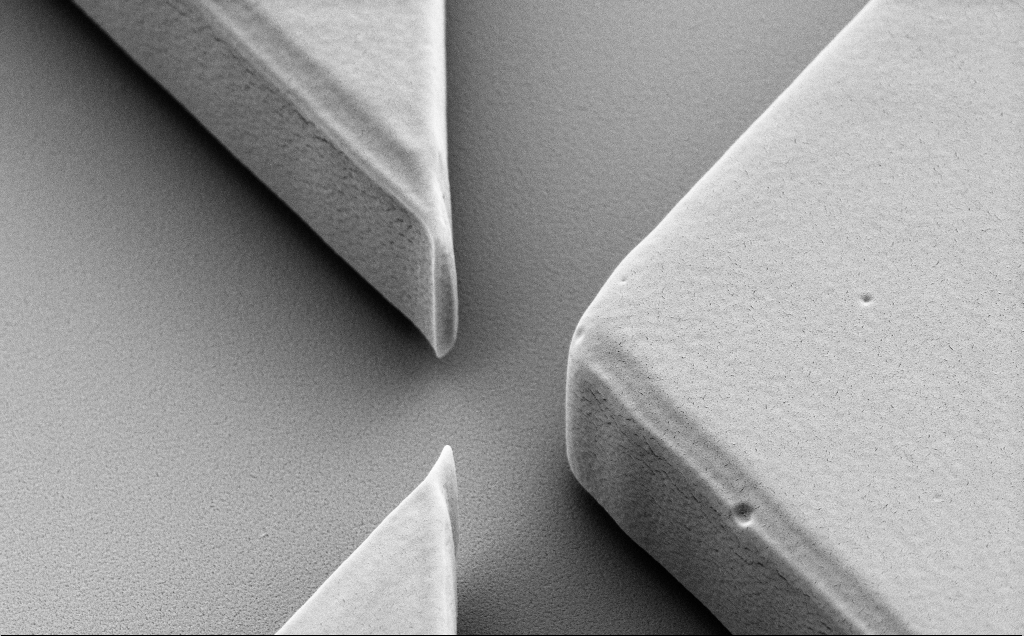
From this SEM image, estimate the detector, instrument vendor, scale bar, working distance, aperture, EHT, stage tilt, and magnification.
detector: SE2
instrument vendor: Zeiss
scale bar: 2000 nm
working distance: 9 mm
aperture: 30 µm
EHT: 5 kV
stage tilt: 40°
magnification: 15 K X